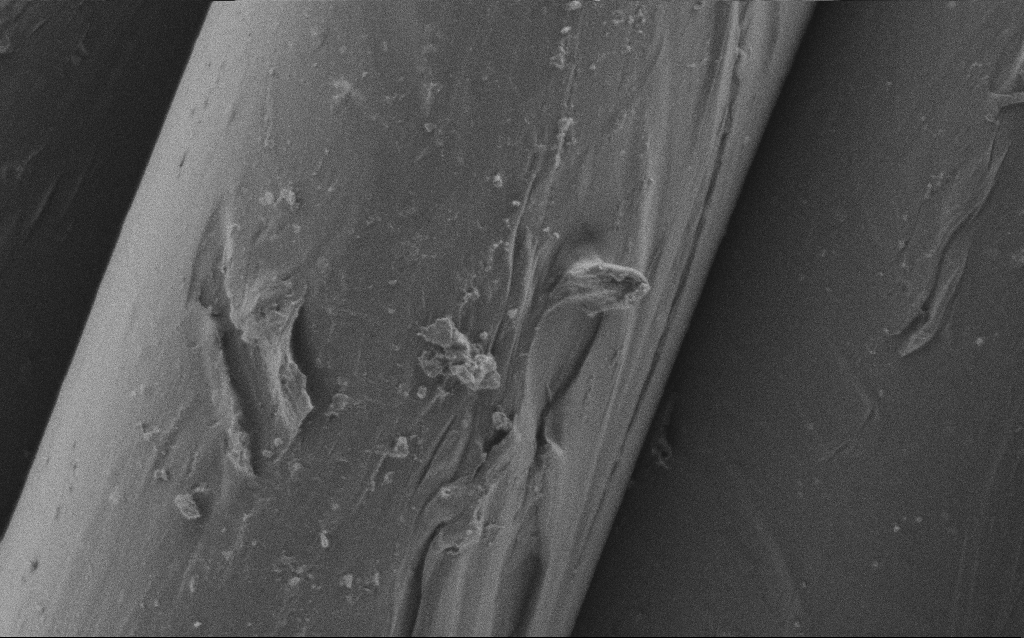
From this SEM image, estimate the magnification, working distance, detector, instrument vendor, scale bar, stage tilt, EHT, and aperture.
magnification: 8.82 K X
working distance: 4 mm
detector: SE2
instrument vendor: Zeiss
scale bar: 2000 nm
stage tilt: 0°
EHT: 1 kV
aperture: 30 µm